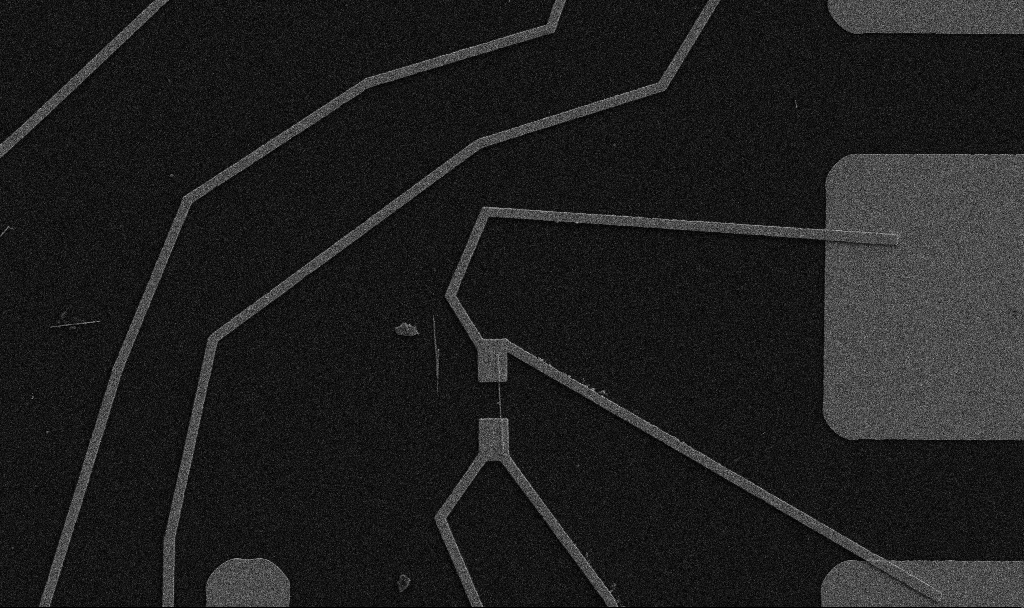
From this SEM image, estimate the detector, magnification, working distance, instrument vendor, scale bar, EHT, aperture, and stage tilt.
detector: SE2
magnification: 5 K X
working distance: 10.7 mm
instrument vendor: Zeiss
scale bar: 10000 nm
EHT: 5 kV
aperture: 30 µm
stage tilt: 0°